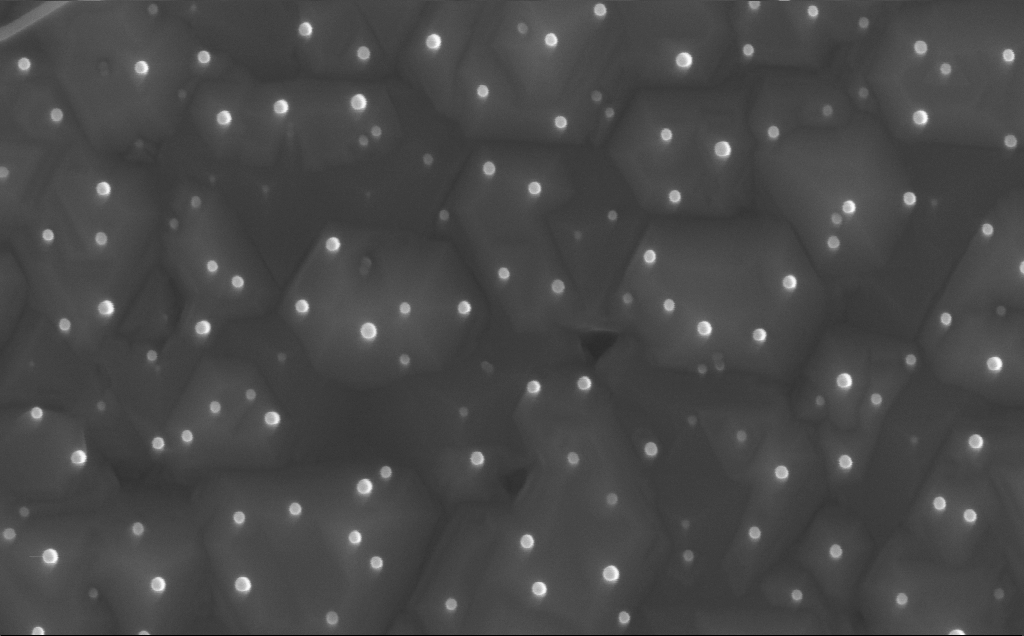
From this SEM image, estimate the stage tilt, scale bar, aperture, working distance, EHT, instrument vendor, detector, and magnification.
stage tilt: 0°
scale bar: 200 nm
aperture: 30 µm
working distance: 6 mm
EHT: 10 kV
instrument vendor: Zeiss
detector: InLens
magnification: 150 K X